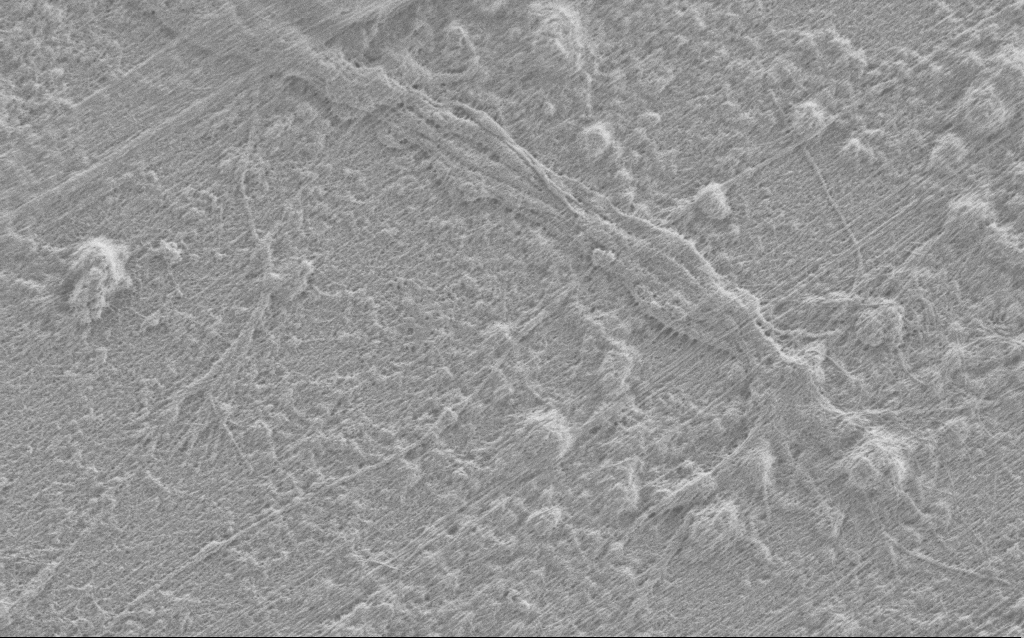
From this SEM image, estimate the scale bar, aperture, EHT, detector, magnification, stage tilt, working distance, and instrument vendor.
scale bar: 2000 nm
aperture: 30 µm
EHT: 0.9 kV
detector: SE2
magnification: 10 K X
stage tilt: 0°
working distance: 4 mm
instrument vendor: Zeiss